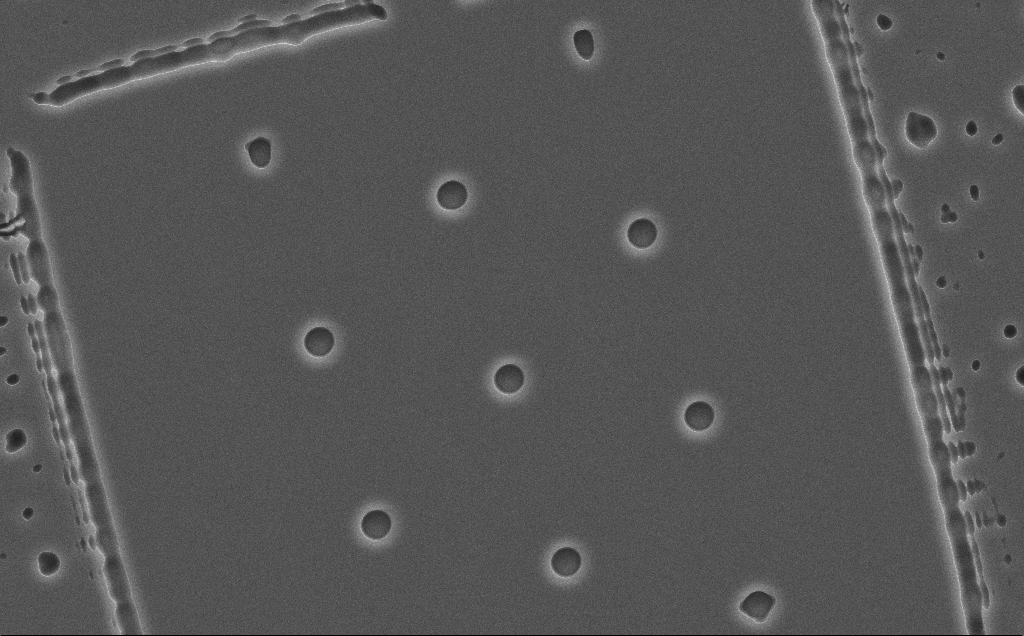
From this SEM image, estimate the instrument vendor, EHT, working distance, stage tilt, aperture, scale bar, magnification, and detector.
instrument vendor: Zeiss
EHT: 10 kV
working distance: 12 mm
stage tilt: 0°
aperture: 30 µm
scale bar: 10000 nm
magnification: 3.52 K X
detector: SE2